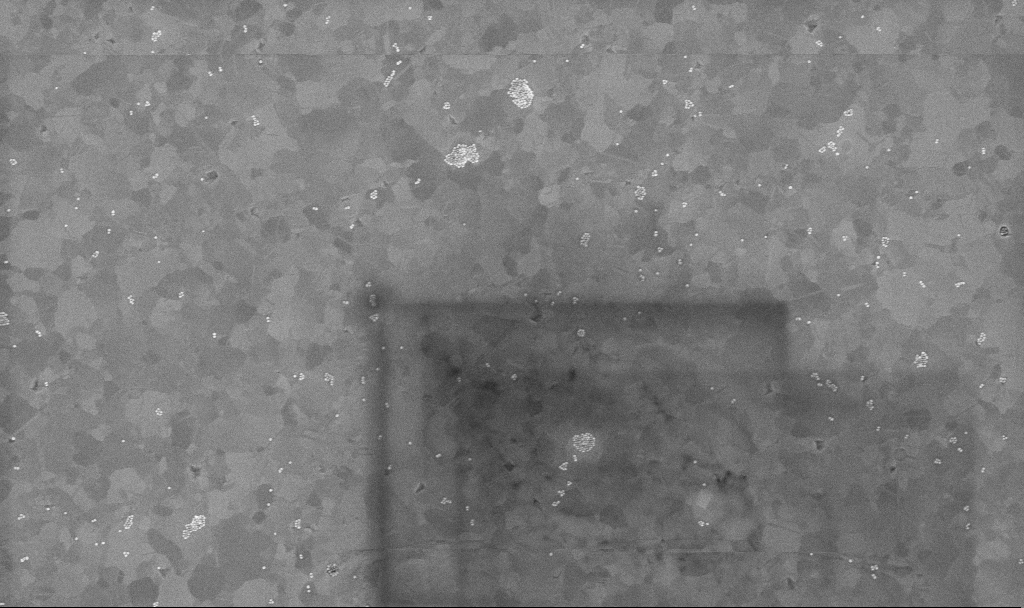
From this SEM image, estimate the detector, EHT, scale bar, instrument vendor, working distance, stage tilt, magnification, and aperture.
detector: InLens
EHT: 10 kV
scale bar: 1000 nm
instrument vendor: Zeiss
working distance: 3.4 mm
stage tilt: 0°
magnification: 50 K X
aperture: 30 µm